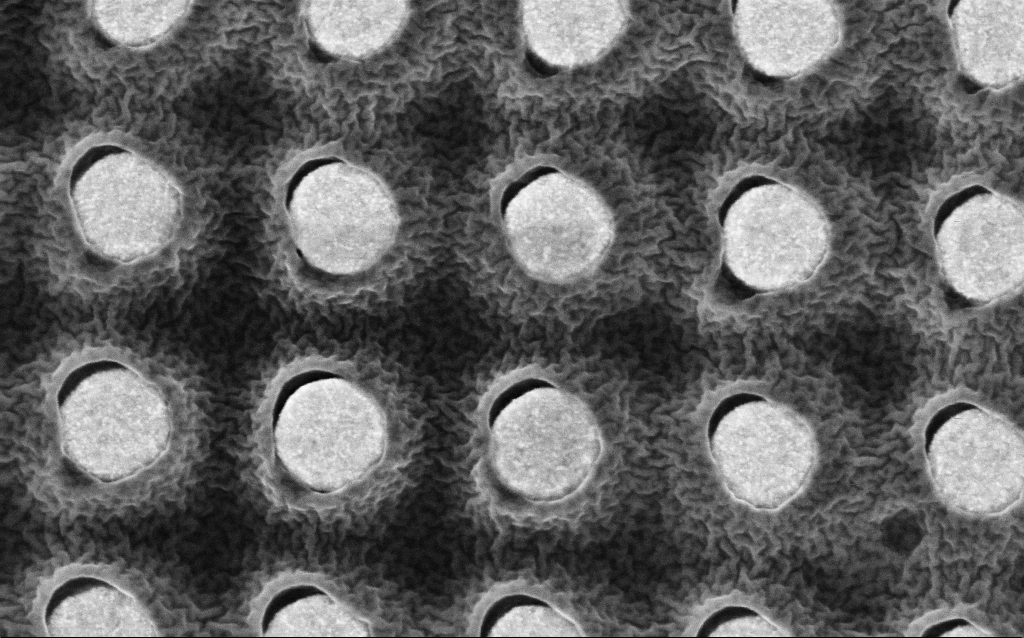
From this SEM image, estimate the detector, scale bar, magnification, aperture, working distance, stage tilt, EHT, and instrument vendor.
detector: InLens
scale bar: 200 nm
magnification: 160.73 K X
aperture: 30 µm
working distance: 3.1 mm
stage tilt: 0°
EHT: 2 kV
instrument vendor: Zeiss